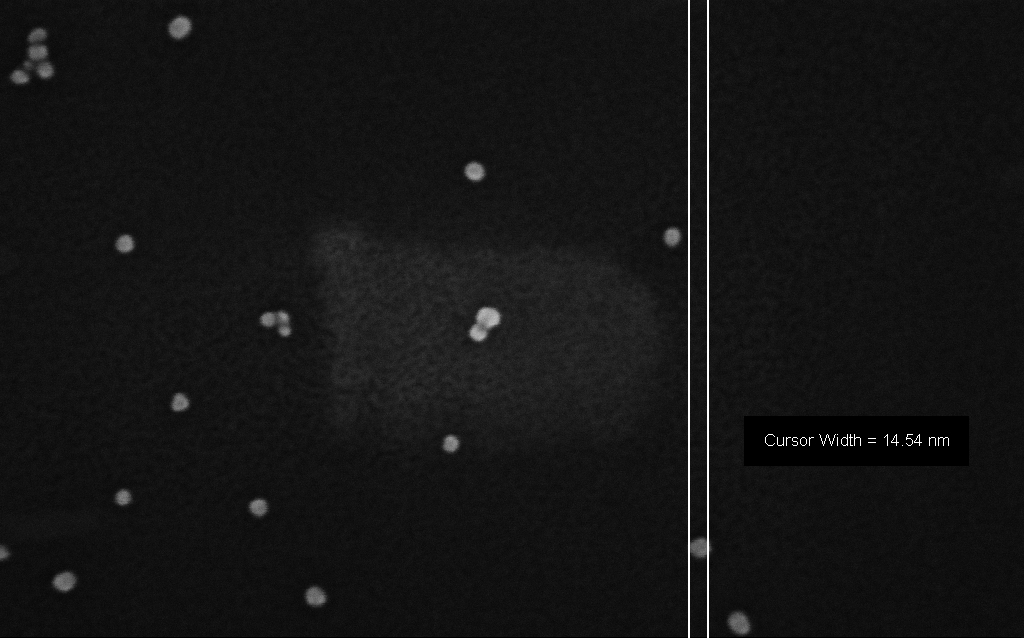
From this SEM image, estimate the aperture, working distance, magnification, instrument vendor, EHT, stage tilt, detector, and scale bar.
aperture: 30 µm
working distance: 2.7 mm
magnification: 479.74 K X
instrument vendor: Zeiss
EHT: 8 kV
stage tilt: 0°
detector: InLens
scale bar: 100 nm